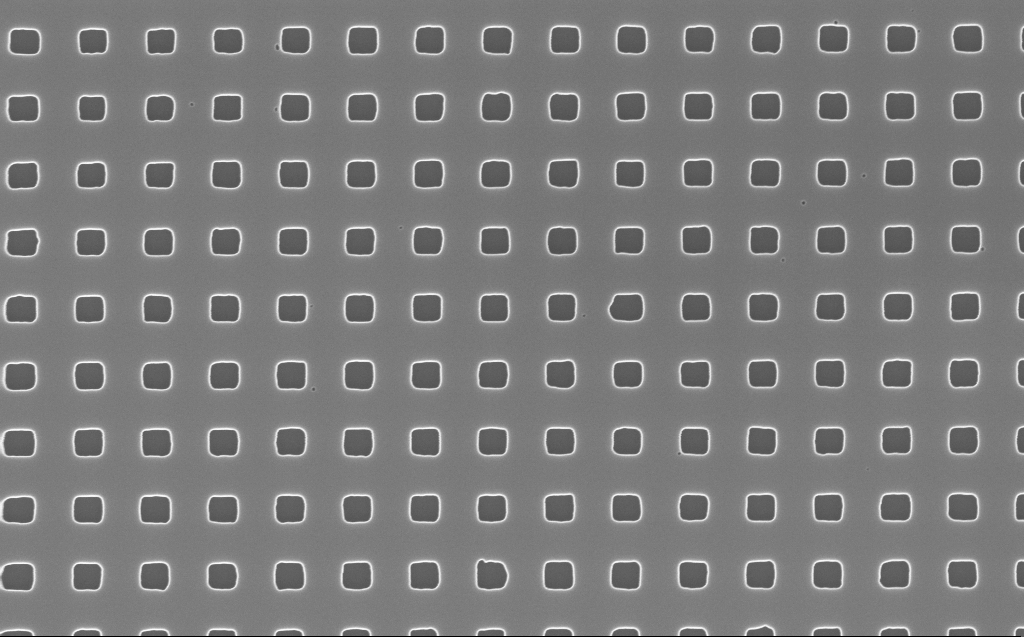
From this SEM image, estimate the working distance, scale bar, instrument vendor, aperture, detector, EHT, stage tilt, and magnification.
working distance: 5 mm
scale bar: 1000 nm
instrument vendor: Zeiss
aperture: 30 µm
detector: InLens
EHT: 10 kV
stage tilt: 0°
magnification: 50 K X